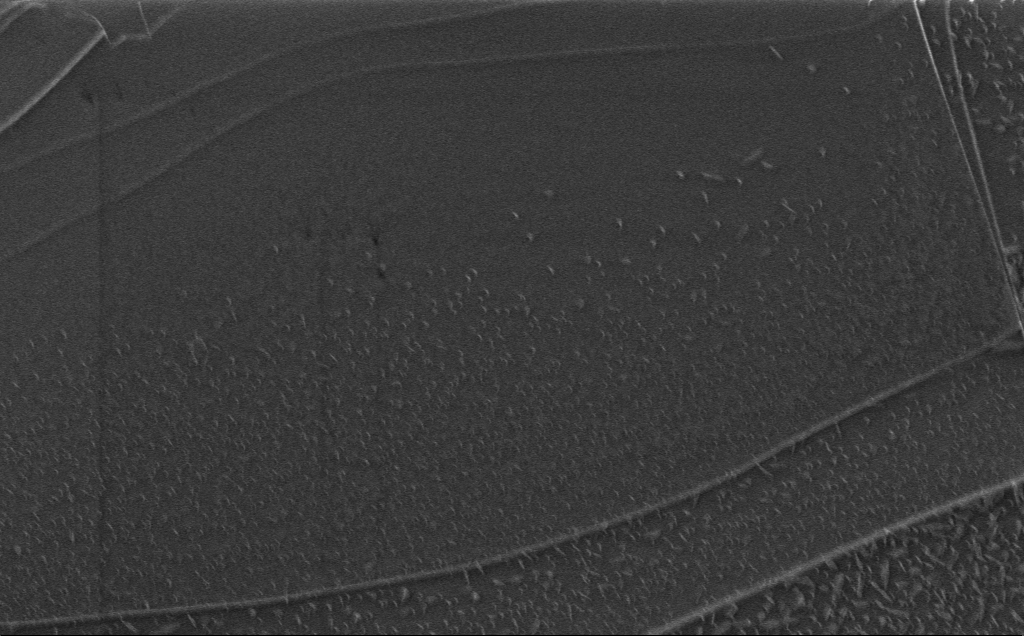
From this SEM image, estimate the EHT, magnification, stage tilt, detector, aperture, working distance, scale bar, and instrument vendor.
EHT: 1 kV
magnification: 5.43 K X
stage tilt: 0°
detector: InLens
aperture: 30 µm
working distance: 3 mm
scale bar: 10000 nm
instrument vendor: Zeiss